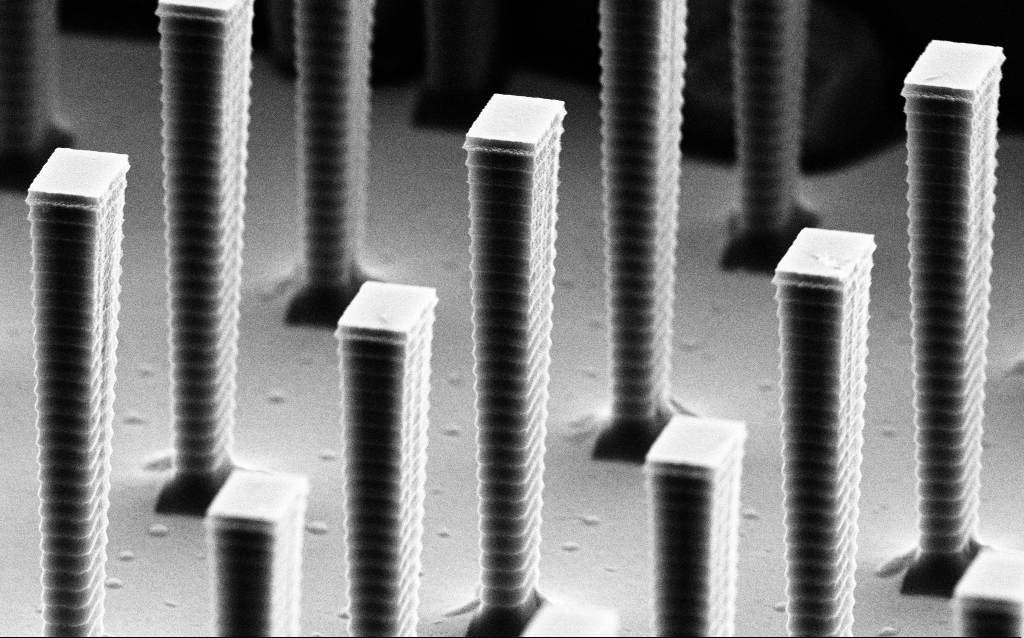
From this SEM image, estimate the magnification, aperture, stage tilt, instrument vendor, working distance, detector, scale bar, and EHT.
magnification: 15.63 K X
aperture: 30 µm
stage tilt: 70°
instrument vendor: Zeiss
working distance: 8.5 mm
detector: SE2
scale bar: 1000 nm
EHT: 8 kV